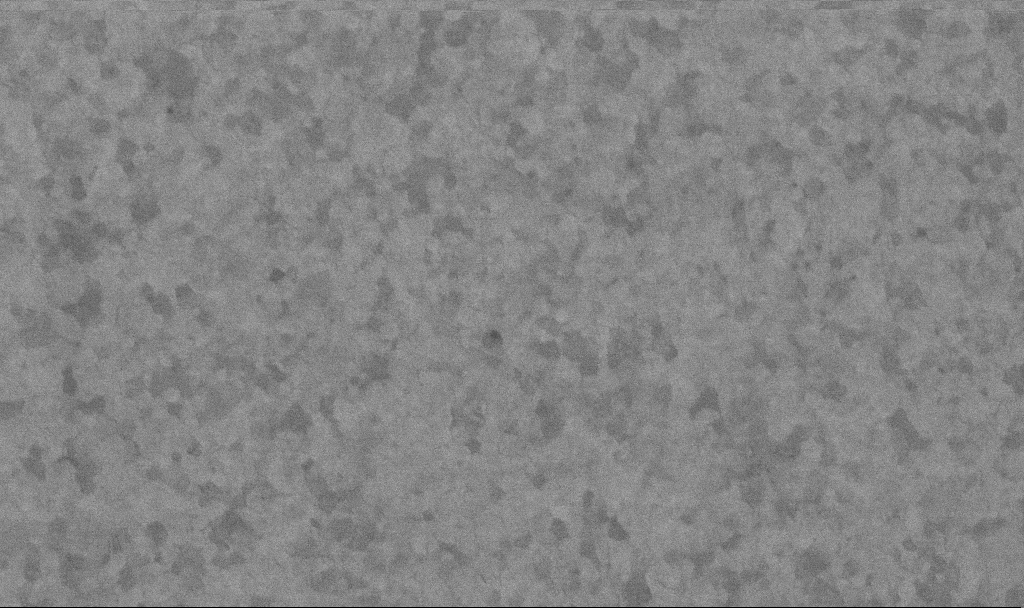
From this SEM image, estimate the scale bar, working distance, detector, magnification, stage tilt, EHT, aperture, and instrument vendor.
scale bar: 200 nm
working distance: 3.4 mm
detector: InLens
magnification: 100.94 K X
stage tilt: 0°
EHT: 10 kV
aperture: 30 µm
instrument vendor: Zeiss